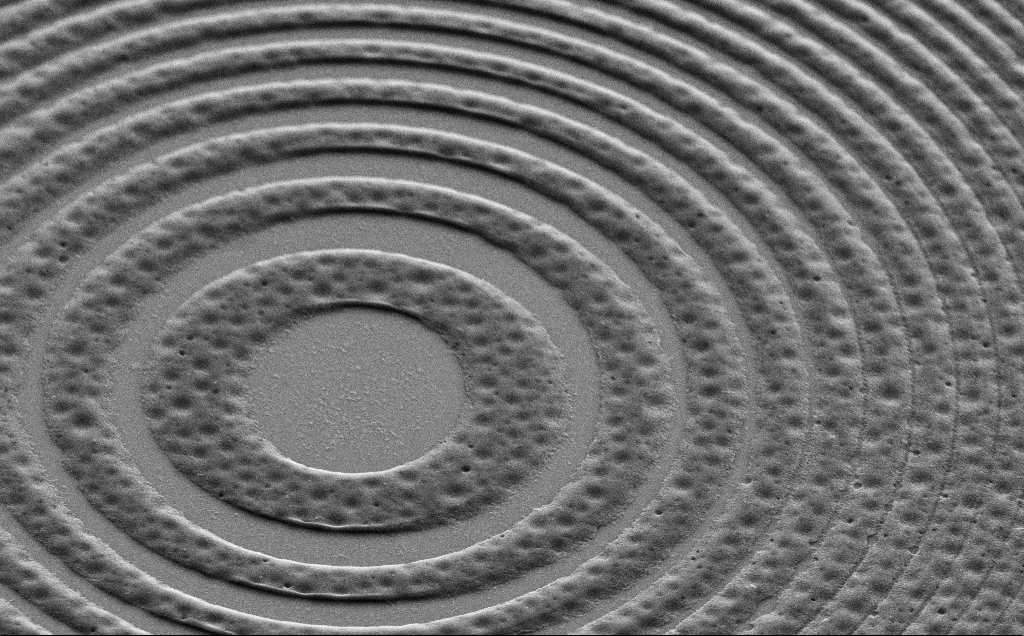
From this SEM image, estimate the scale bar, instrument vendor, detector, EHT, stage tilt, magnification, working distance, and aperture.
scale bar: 2000 nm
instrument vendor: Zeiss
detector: SE2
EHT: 5 kV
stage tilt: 45°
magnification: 12.2 K X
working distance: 7 mm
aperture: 30 µm